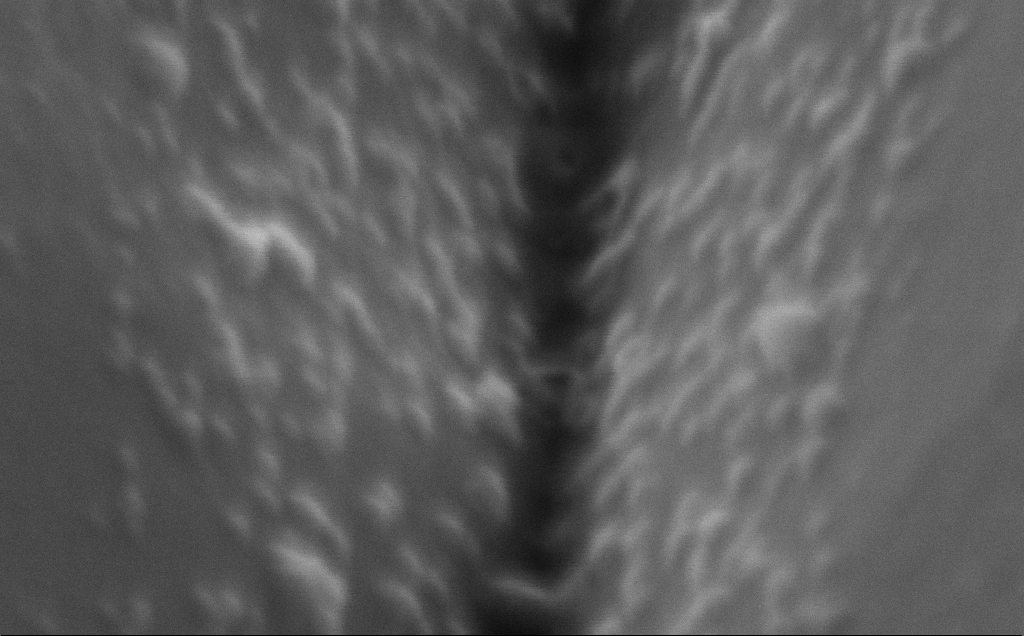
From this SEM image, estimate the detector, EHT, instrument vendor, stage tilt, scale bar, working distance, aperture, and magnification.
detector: SE2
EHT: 5 kV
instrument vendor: Zeiss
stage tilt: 50°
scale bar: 100 nm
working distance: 10 mm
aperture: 30 µm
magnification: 408.5 K X